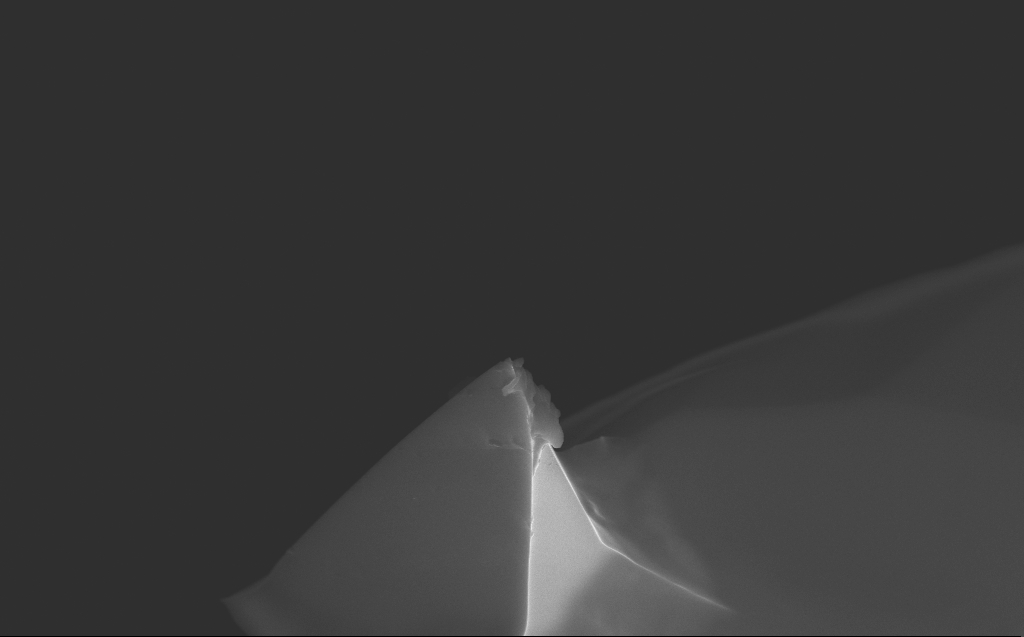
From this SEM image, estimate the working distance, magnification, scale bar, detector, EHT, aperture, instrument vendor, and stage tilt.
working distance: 8 mm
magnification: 11.19 K X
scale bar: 2000 nm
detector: InLens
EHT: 10 kV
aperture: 30 µm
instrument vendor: Zeiss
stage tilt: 35.7°